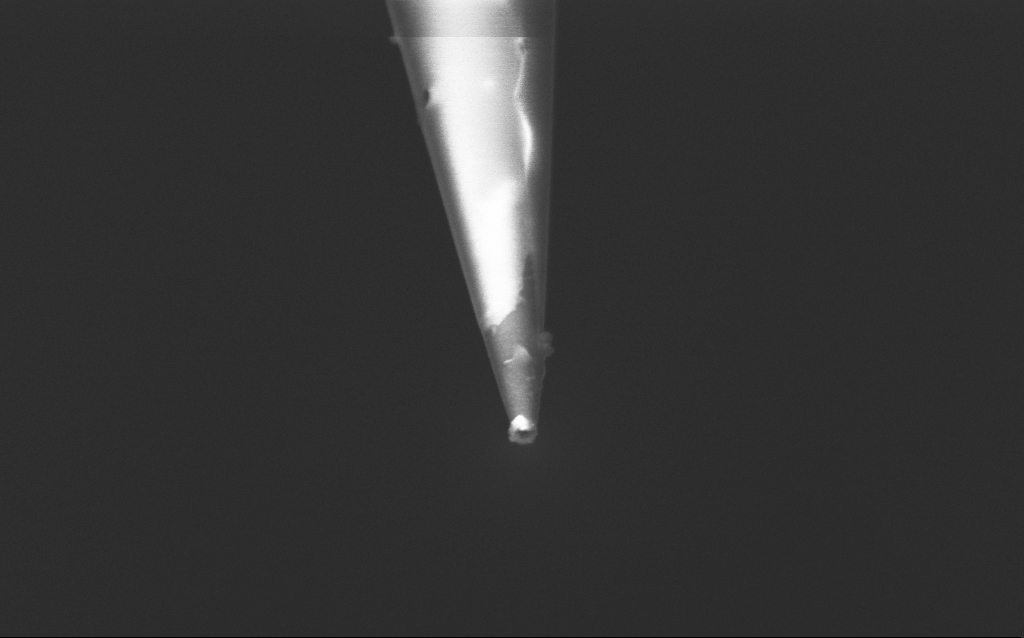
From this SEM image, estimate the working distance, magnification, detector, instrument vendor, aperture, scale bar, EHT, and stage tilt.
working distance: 5 mm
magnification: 100 K X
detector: InLens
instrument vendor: Zeiss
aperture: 30 µm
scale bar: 200 nm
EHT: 2.5 kV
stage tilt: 45°